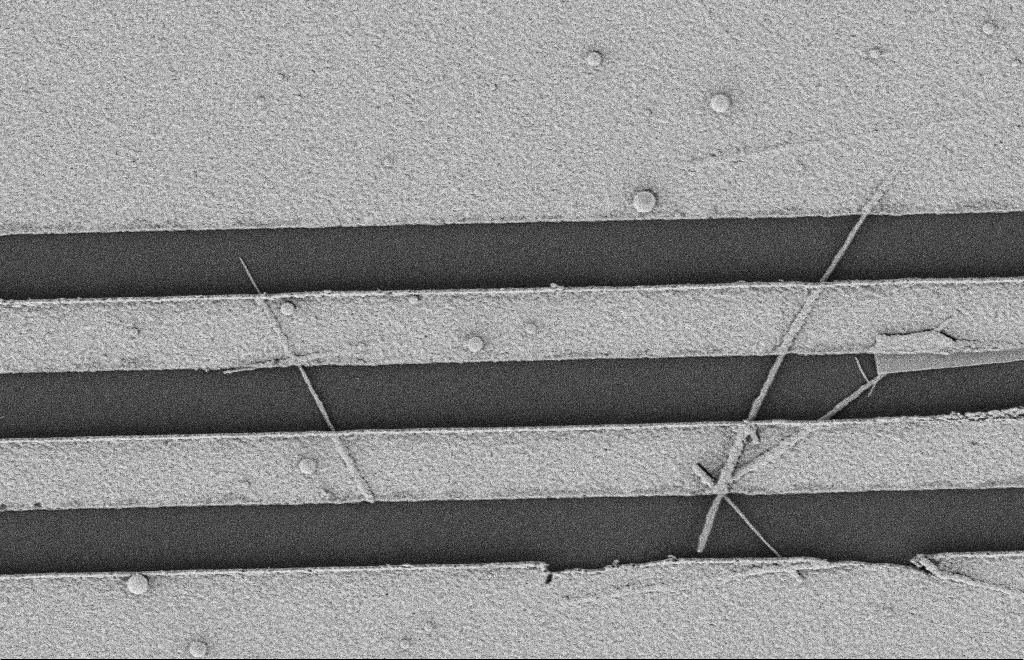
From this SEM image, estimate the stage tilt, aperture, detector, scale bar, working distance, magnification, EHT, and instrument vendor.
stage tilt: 0°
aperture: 20 µm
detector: SE2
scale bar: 2000 nm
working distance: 12 mm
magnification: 12.29 K X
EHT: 2 kV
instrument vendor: Zeiss